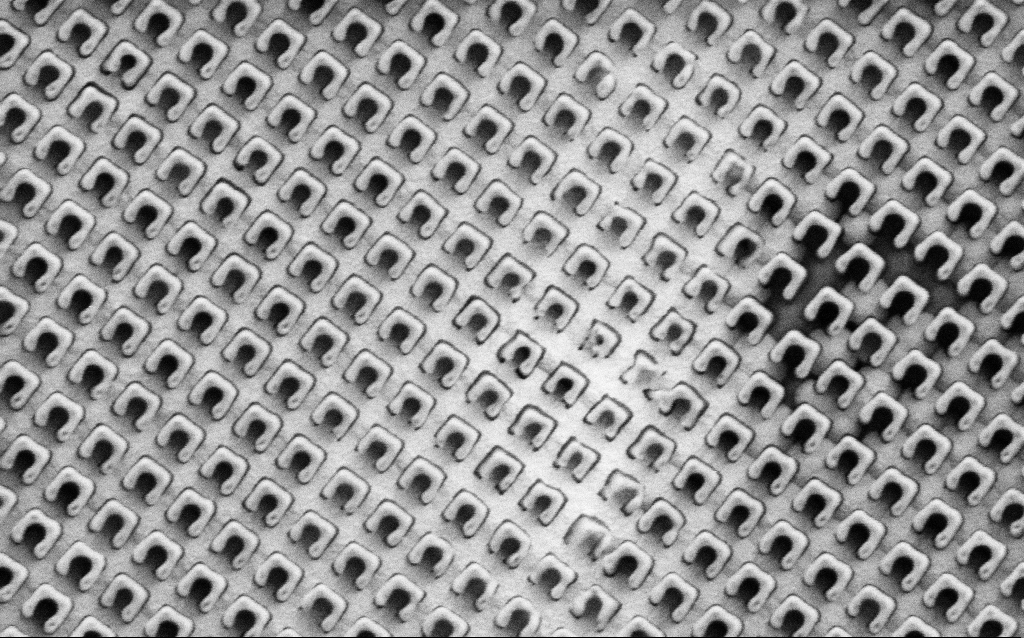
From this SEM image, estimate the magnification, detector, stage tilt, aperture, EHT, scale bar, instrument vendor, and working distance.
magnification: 43.24 K X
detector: SE2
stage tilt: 0°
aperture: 30 µm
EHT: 1.5 kV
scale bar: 1000 nm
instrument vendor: Zeiss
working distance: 8 mm